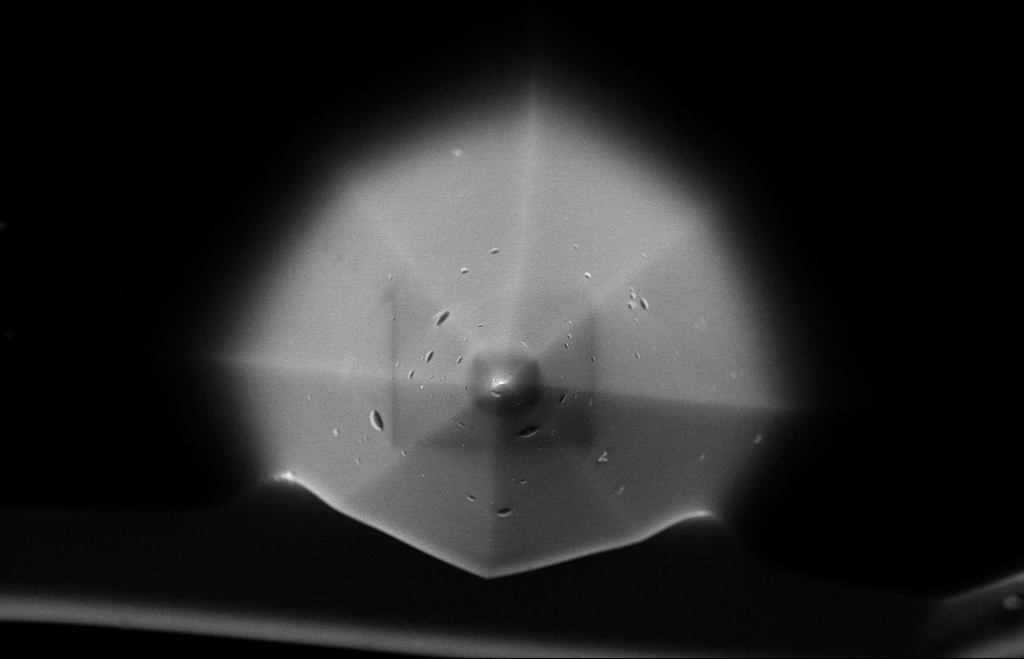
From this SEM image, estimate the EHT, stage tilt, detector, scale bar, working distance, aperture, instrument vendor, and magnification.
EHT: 10 kV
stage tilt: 0°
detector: InLens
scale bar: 1000 nm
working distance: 8 mm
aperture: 30 µm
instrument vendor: Zeiss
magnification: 15.61 K X